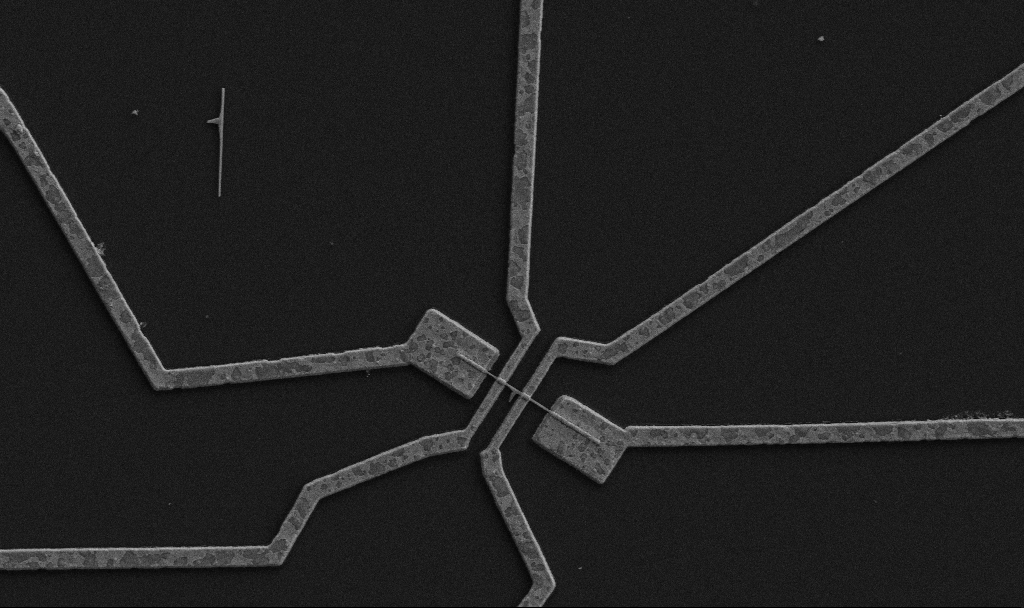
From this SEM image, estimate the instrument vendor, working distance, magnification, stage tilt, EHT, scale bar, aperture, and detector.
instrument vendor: Zeiss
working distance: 9.7 mm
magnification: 10 K X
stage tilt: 0°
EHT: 5 kV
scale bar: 2000 nm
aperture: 30 µm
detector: SE2